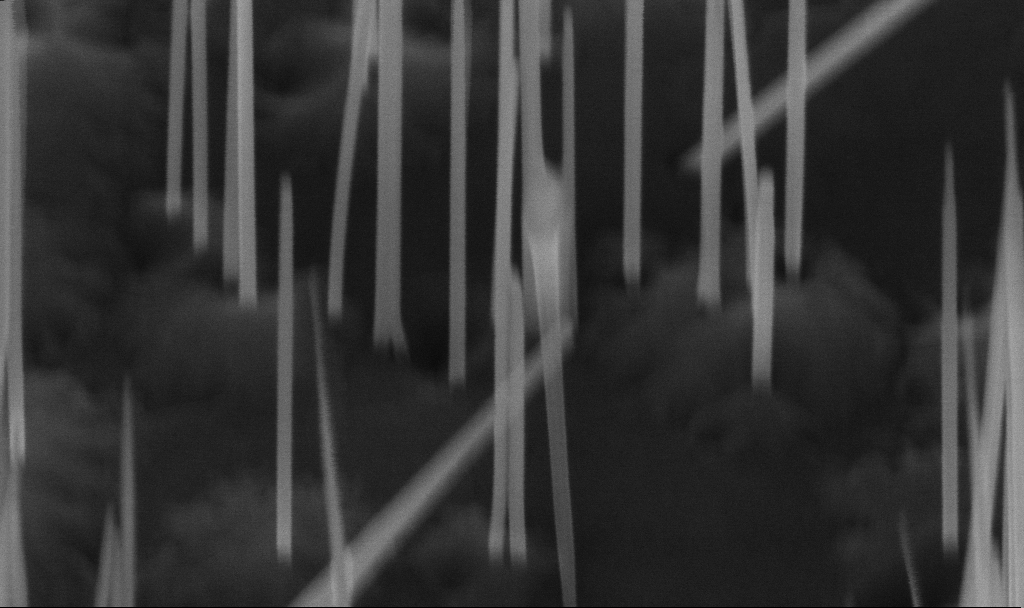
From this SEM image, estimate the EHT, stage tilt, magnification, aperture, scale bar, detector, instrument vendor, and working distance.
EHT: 10 kV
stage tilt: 45°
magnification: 220.34 K X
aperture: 30 µm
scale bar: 100 nm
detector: InLens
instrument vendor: Zeiss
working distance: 5.6 mm